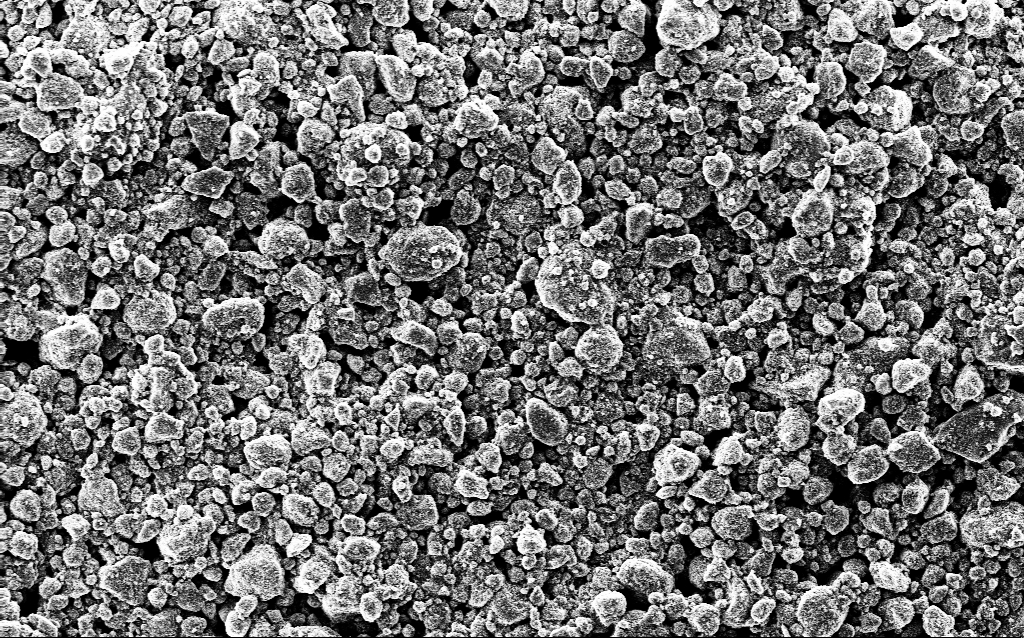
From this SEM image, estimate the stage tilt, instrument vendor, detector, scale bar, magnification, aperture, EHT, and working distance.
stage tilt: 0°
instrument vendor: Zeiss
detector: InLens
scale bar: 10000 nm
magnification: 3.3 K X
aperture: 30 µm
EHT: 5 kV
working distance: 1.6 mm